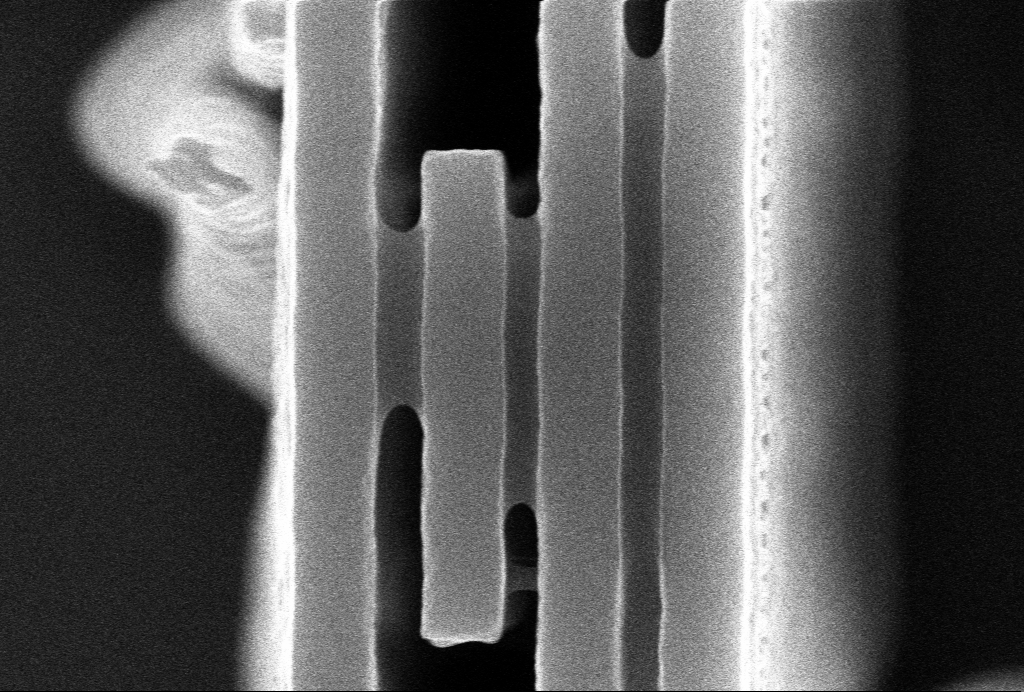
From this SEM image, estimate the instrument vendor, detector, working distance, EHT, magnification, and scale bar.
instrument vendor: Zeiss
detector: InLens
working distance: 5 mm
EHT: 10 kV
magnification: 60.28 K X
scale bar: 300 nm